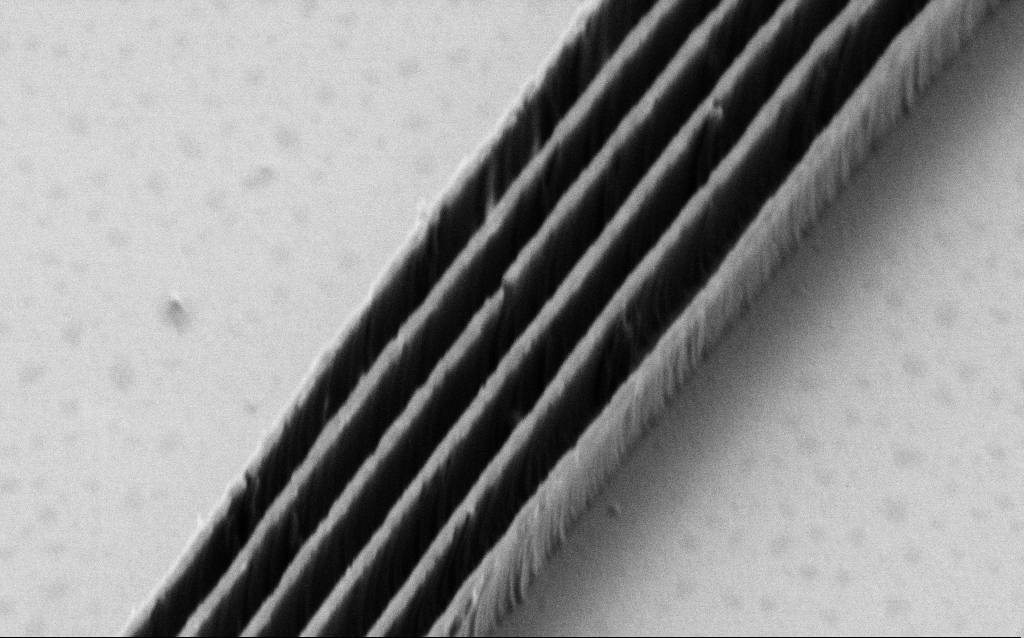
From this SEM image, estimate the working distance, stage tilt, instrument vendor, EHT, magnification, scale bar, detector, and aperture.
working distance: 7 mm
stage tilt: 45°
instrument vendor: Zeiss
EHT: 1.5 kV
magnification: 44.28 K X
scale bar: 1000 nm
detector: SE2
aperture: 30 µm